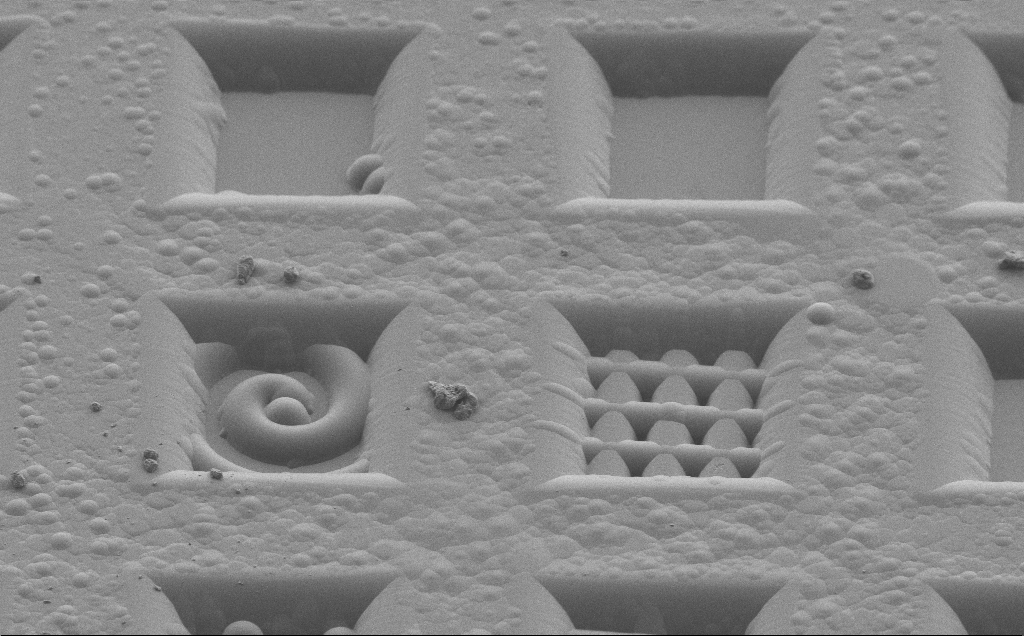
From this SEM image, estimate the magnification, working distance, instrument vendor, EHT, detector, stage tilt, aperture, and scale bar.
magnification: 0.994 K X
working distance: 7 mm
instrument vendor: Zeiss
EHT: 1.3 kV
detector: SE2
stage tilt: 45°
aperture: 30 µm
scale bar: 20000 nm